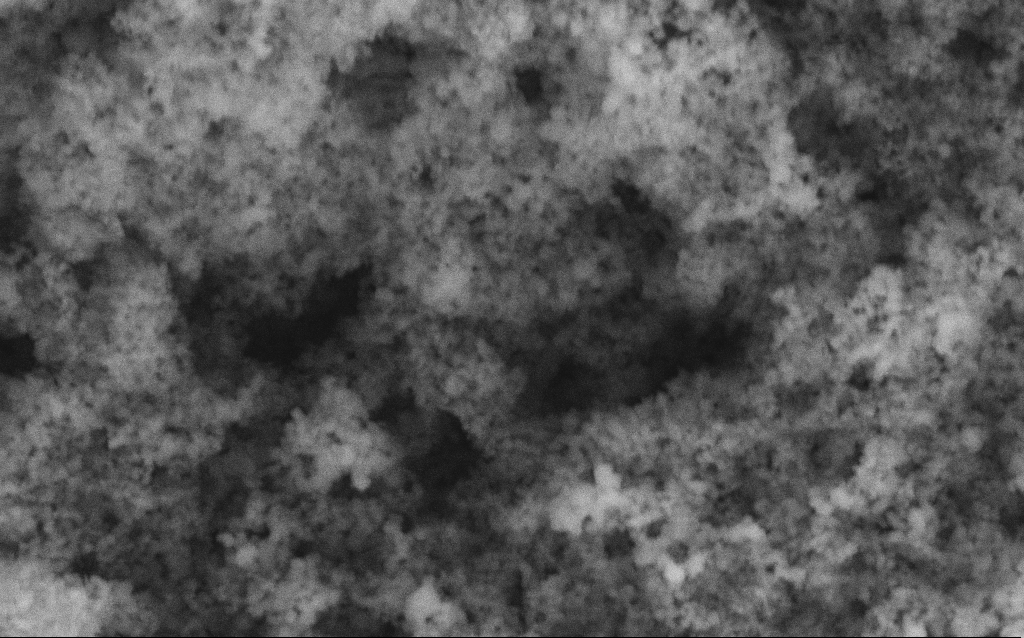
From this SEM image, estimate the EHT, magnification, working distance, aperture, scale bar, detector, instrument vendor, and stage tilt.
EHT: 5 kV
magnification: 114.64 K X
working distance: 4.2 mm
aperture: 30 µm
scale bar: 200 nm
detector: SE2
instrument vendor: Zeiss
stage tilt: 0°